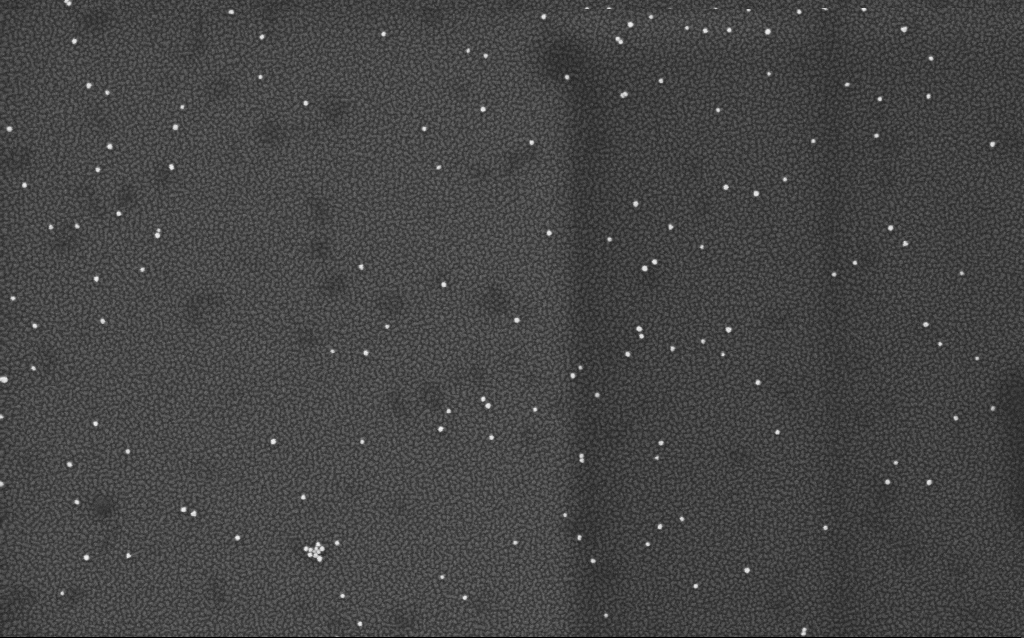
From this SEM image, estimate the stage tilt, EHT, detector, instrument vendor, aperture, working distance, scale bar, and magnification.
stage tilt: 0°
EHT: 2 kV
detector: InLens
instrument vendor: Zeiss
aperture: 30 µm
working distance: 1.7 mm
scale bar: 200 nm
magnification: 100 K X